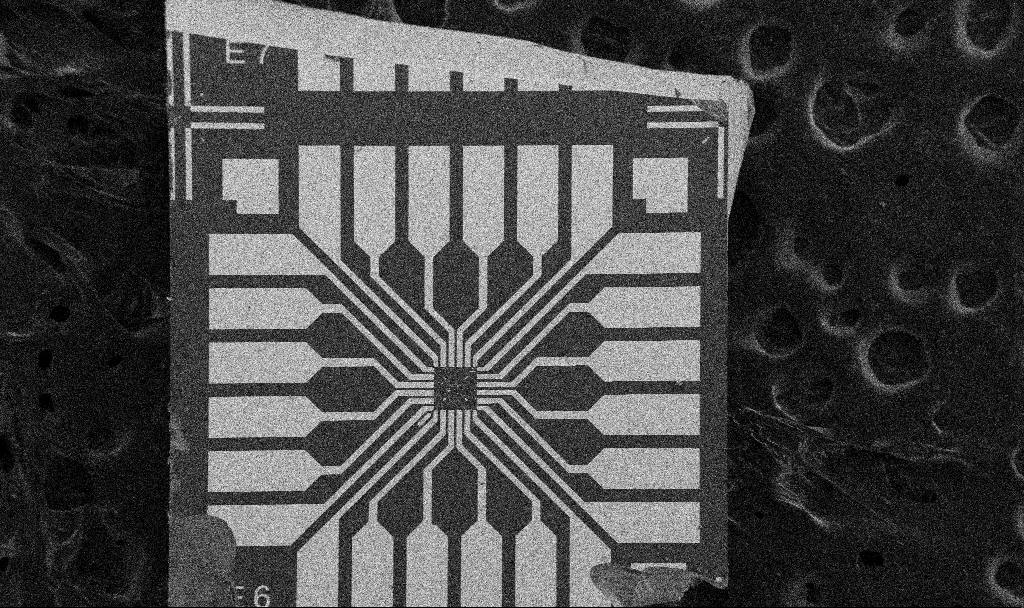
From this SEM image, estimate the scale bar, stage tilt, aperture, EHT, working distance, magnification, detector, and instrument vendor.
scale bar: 200000 nm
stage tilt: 0°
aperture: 30 µm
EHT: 5 kV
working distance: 8.7 mm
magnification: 0.1 K X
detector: SE2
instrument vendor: Zeiss